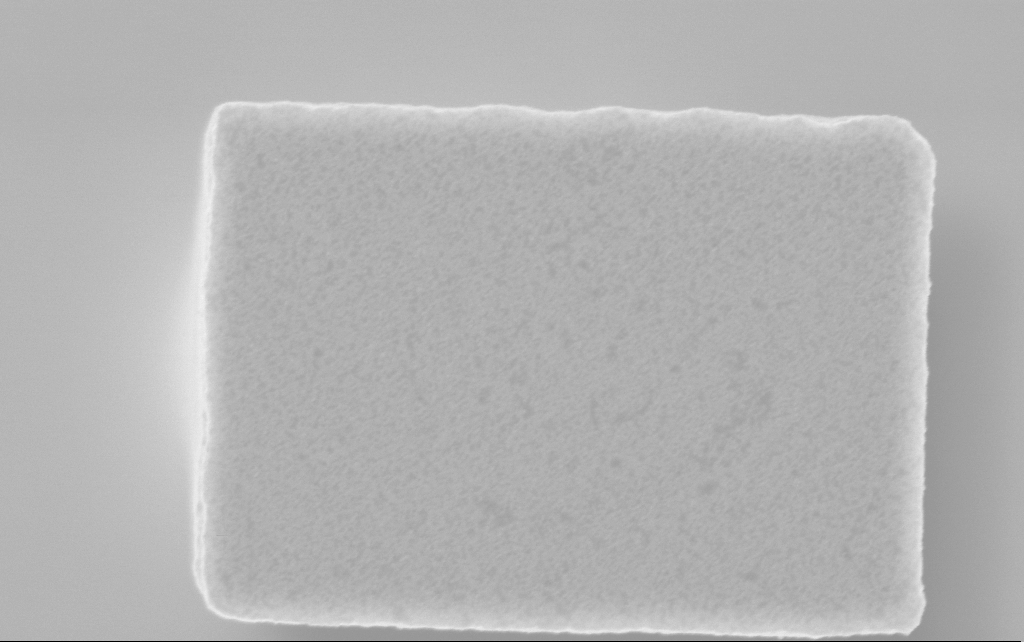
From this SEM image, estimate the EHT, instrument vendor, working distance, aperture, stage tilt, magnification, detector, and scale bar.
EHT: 3 kV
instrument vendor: Zeiss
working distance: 2.5 mm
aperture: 30 µm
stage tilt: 0°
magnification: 88.28 K X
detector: InLens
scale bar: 200 nm